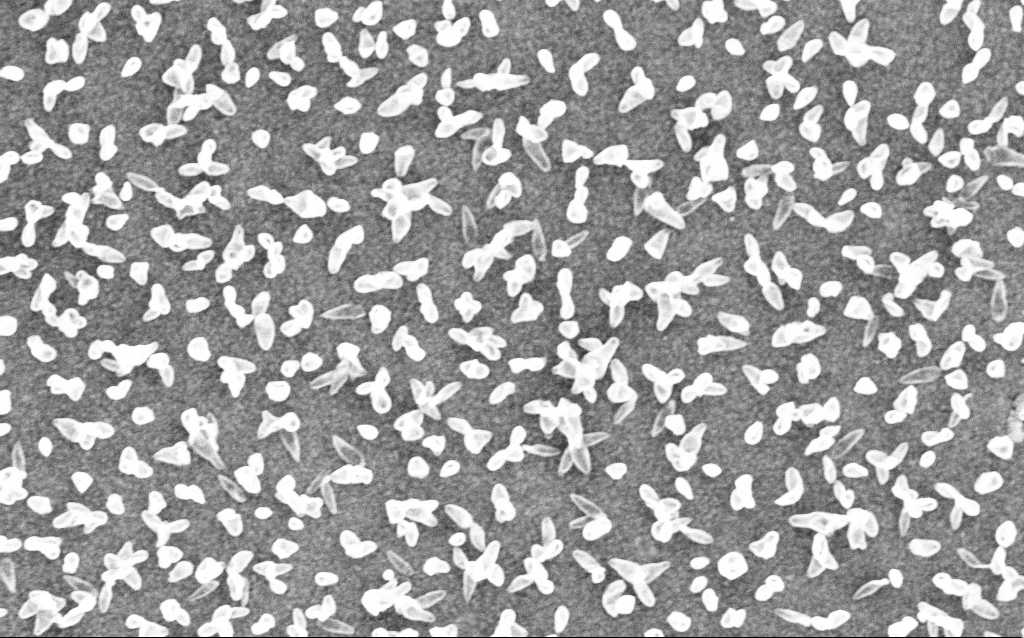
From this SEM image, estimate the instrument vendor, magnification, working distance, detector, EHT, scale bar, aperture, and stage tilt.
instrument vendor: Zeiss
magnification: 44.01 K X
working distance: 6 mm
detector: InLens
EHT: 6 kV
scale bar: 1000 nm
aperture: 30 µm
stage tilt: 0°